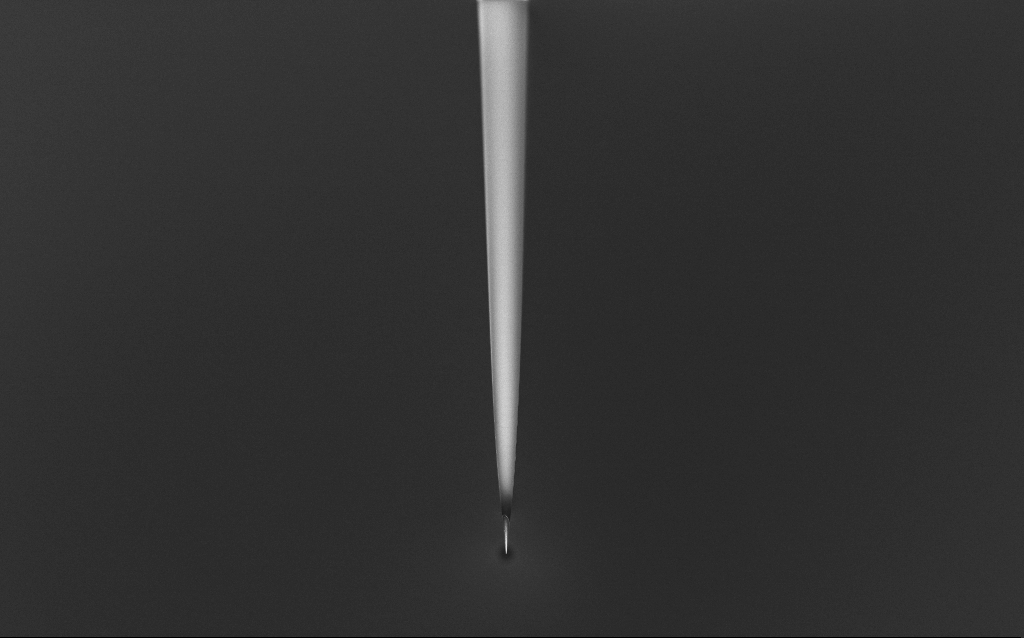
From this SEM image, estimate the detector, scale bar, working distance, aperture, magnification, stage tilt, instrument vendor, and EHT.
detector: InLens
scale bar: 100000 nm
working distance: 6 mm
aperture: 30 µm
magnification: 0.5 K X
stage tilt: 45°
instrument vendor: Zeiss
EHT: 1 kV